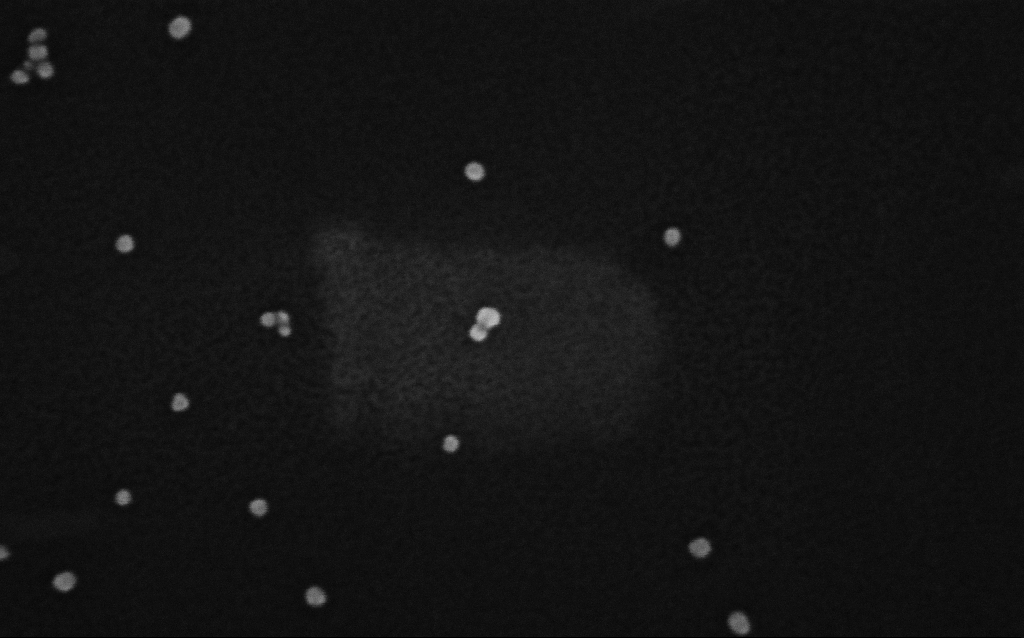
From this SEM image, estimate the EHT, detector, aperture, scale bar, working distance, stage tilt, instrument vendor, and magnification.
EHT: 8 kV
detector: InLens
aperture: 30 µm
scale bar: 100 nm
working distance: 2.7 mm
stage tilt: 0°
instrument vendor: Zeiss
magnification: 479.74 K X